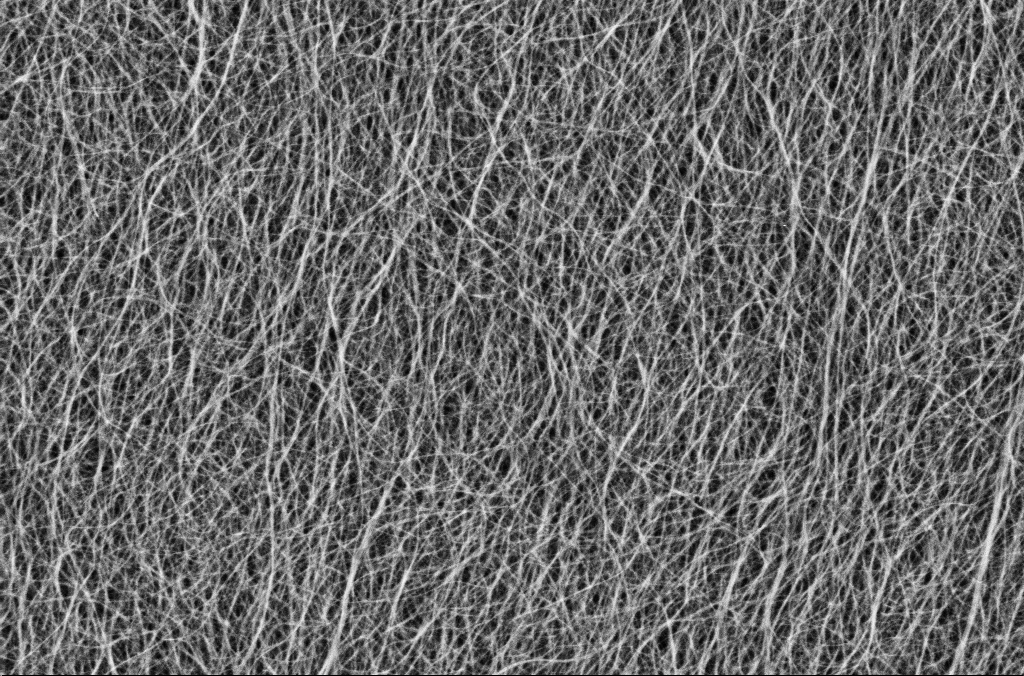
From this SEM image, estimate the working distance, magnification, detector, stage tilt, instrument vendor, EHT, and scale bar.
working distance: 5.7 mm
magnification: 50 K X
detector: SE2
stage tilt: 0°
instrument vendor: Zeiss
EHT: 1.8 kV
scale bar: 1000 nm